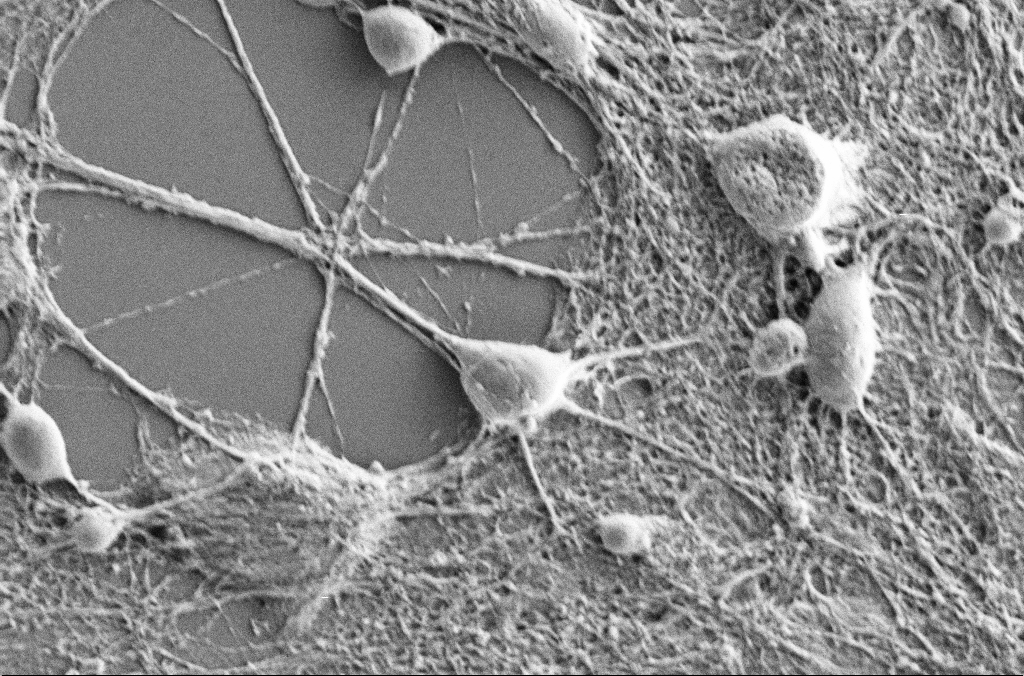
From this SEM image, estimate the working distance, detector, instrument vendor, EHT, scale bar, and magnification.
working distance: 3.7 mm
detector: SE2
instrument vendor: Zeiss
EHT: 7 kV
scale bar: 10000 nm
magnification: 2.5 K X